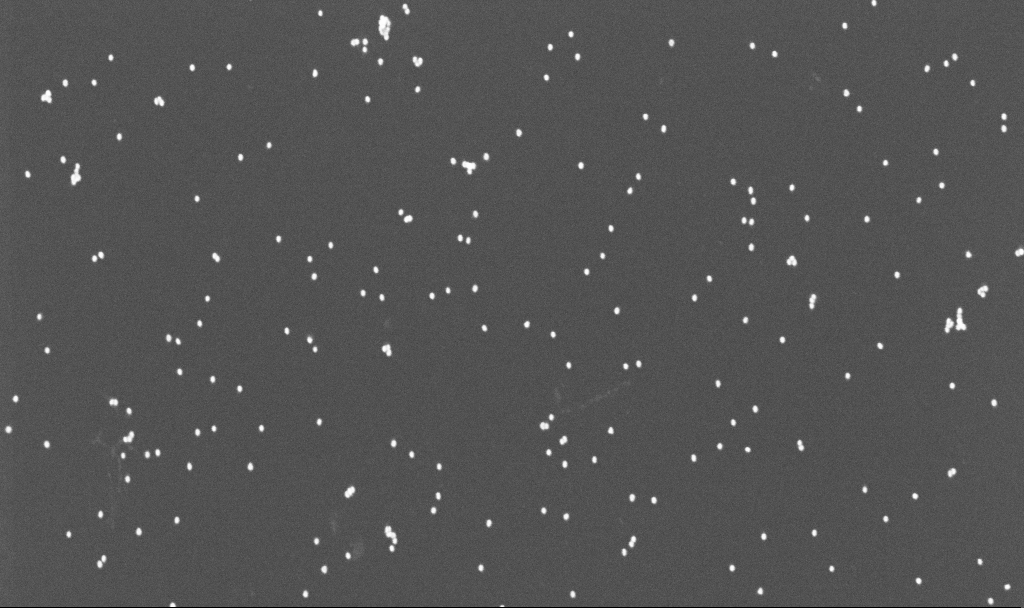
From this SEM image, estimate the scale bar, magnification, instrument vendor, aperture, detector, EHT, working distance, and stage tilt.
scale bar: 1000 nm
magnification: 70 K X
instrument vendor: Zeiss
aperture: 30 µm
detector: InLens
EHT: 10 kV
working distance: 3.3 mm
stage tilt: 0°